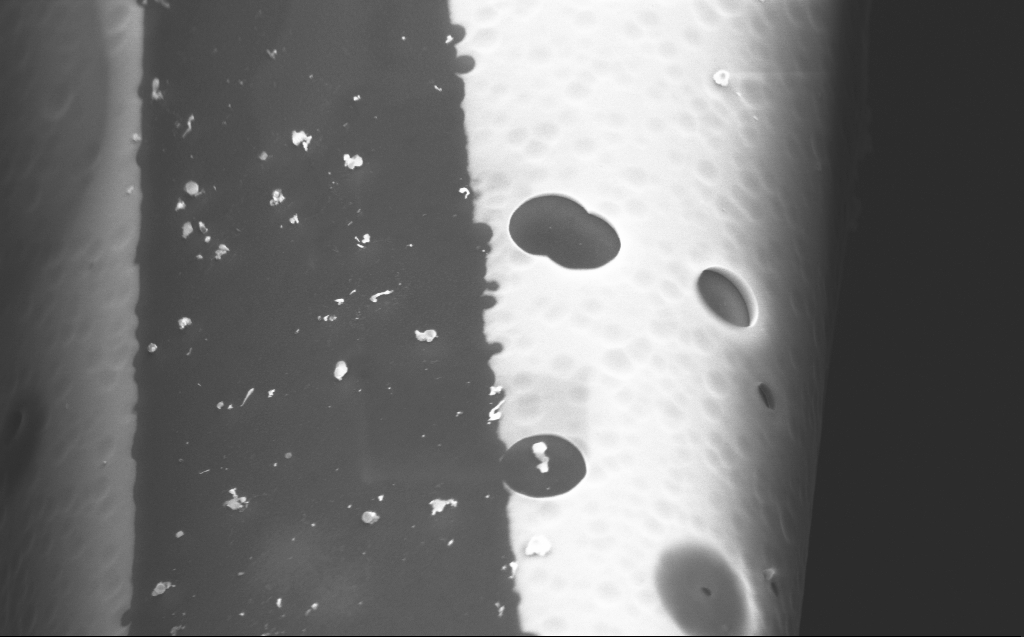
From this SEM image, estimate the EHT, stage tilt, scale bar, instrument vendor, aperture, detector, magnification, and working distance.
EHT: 5 kV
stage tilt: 45°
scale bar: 2000 nm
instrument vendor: Zeiss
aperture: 30 µm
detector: InLens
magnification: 35.9 K X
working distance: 4 mm